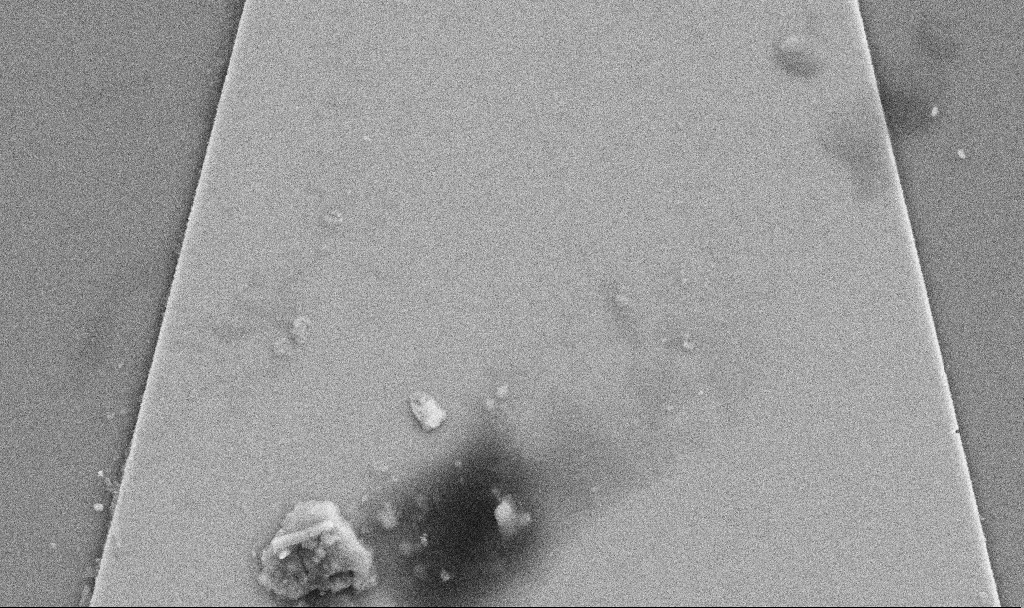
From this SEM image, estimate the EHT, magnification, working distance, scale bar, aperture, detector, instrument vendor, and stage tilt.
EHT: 5 kV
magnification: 26.45 K X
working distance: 10.3 mm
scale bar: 2000 nm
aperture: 30 µm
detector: SE2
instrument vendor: Zeiss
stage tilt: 0°